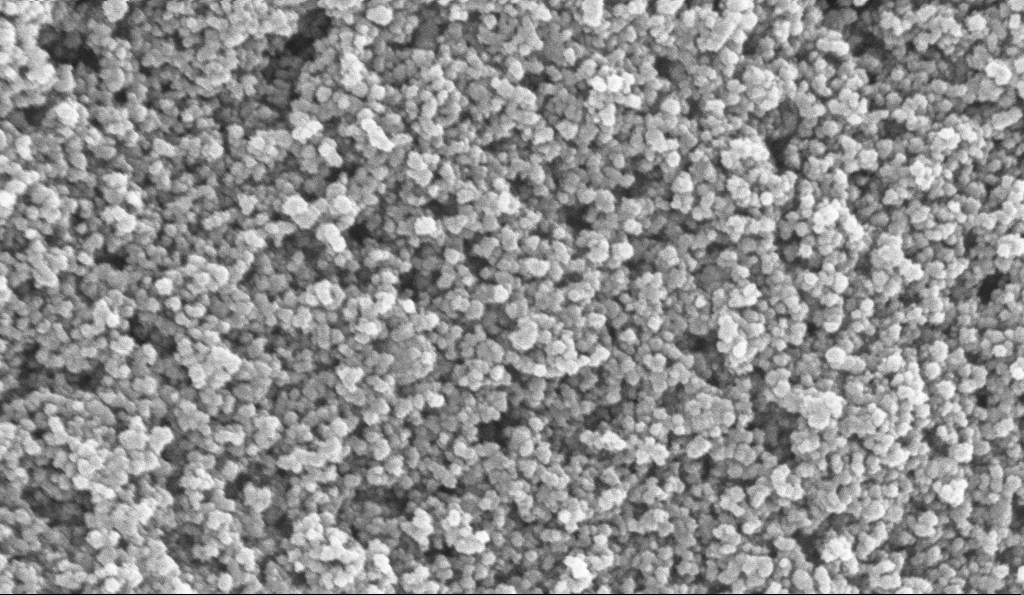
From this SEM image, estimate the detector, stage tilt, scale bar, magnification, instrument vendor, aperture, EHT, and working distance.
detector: InLens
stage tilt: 0°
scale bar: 100 nm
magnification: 135 K X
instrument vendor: Zeiss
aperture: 30 µm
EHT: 10 kV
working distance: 6 mm